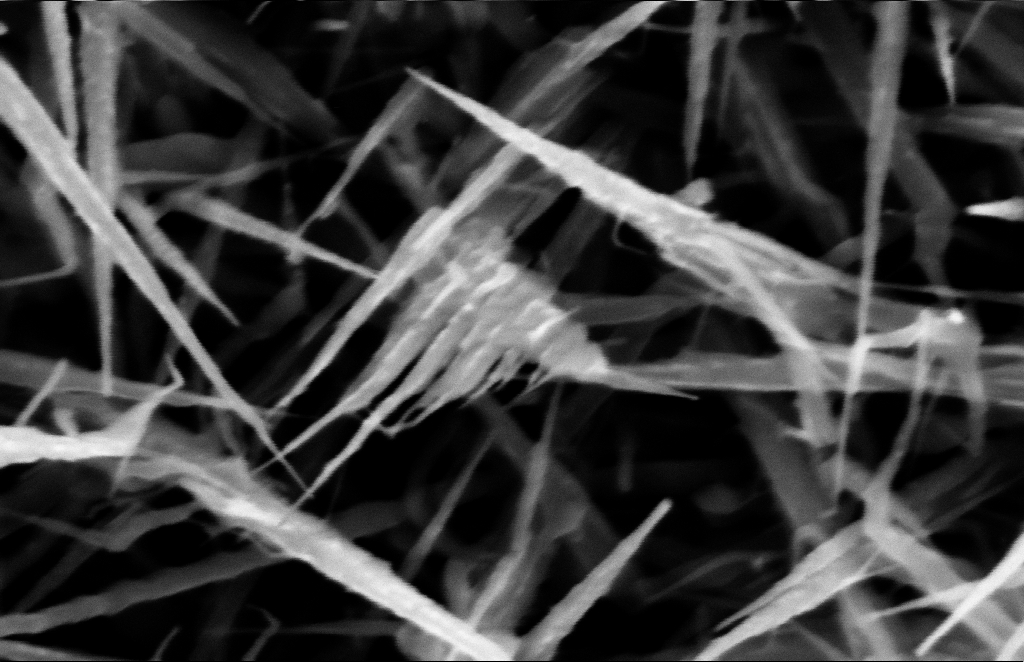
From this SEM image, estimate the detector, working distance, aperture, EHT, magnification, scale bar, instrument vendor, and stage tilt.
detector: InLens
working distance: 10 mm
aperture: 30 µm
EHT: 10 kV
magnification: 248.47 K X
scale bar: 100 nm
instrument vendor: Zeiss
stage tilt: -1.1°